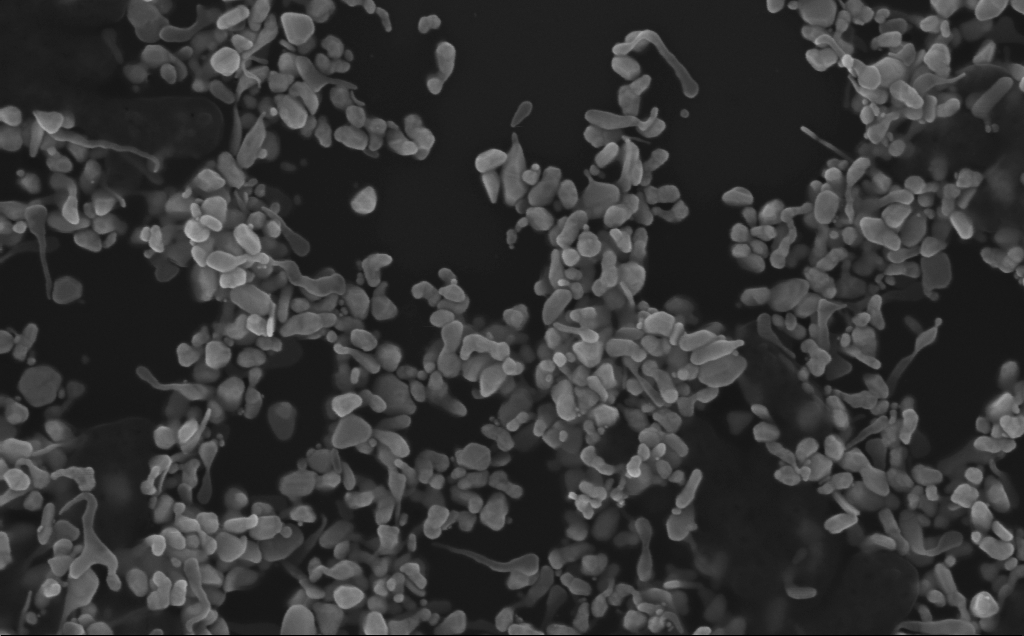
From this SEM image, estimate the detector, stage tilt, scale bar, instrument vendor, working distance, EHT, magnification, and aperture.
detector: InLens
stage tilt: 0°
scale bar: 200 nm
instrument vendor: Zeiss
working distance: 3 mm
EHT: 10 kV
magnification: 140.14 K X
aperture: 30 µm